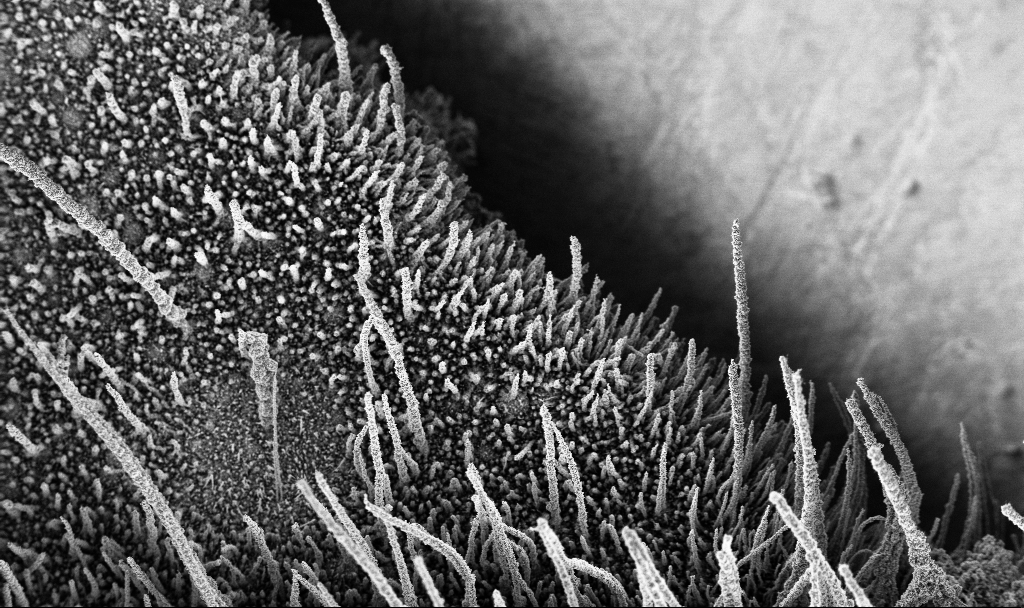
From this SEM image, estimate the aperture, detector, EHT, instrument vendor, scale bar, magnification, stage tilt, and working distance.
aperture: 30 µm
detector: InLens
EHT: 3 kV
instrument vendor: Zeiss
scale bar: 100000 nm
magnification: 0.25 K X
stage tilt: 0°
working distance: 3.8 mm